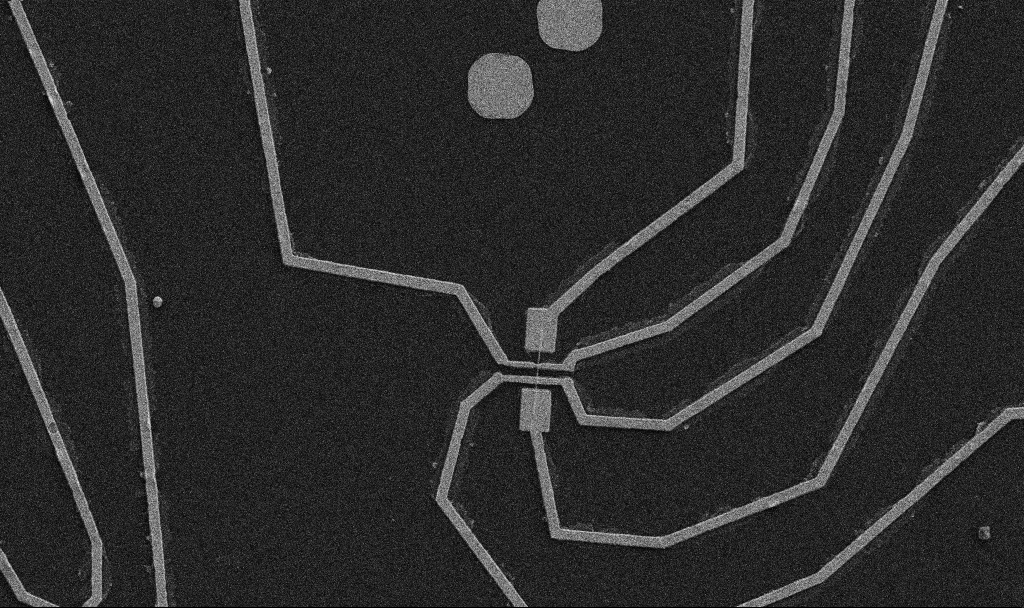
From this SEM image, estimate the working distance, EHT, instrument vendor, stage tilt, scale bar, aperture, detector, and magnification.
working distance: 10.7 mm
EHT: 5 kV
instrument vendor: Zeiss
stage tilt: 0°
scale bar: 10000 nm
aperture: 30 µm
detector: SE2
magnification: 5 K X